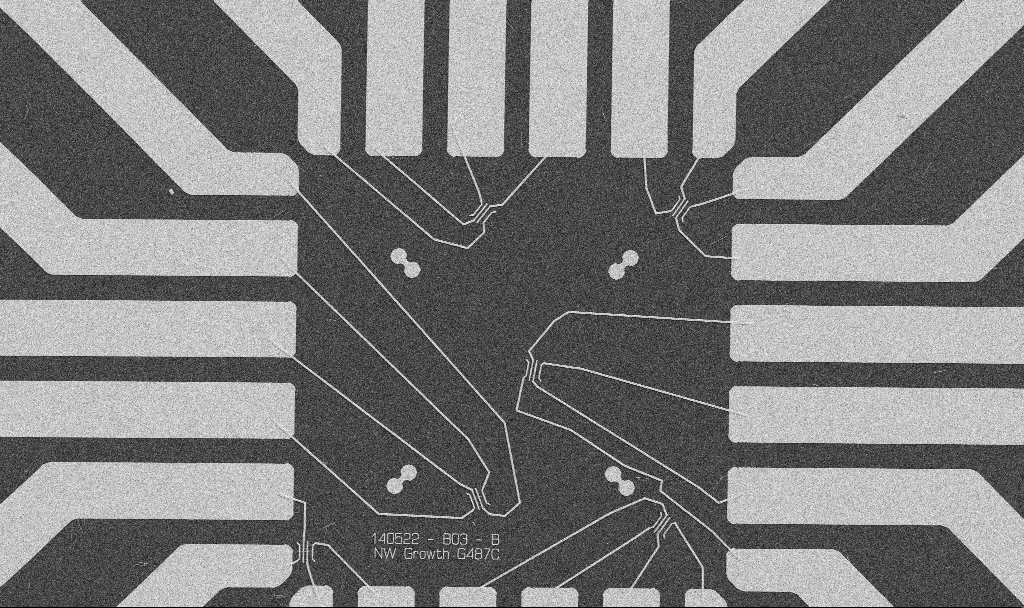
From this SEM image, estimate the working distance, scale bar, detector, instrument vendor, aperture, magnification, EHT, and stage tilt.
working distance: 10.7 mm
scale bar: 20000 nm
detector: SE2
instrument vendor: Zeiss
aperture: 30 µm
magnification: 1 K X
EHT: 5 kV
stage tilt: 0°